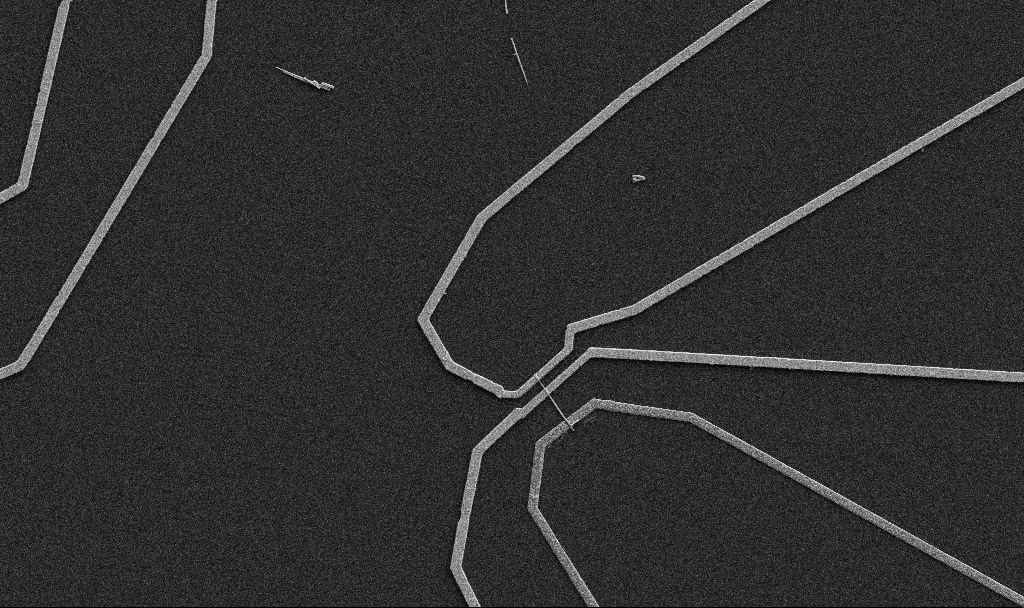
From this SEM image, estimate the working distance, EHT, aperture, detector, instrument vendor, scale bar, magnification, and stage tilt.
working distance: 10.7 mm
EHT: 5 kV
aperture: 30 µm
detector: SE2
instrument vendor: Zeiss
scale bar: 10000 nm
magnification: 5 K X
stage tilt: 0°